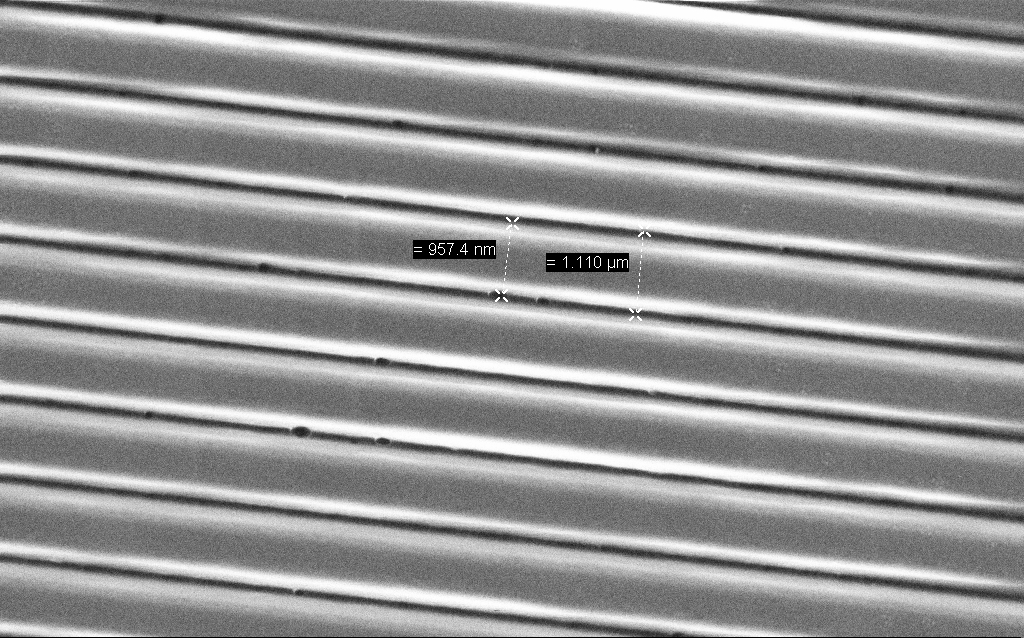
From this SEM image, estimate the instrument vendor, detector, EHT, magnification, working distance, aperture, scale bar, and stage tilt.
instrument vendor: Zeiss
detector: SE2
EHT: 1.5 kV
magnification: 27.93 K X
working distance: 6.5 mm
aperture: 30 µm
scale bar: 2000 nm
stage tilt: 0°